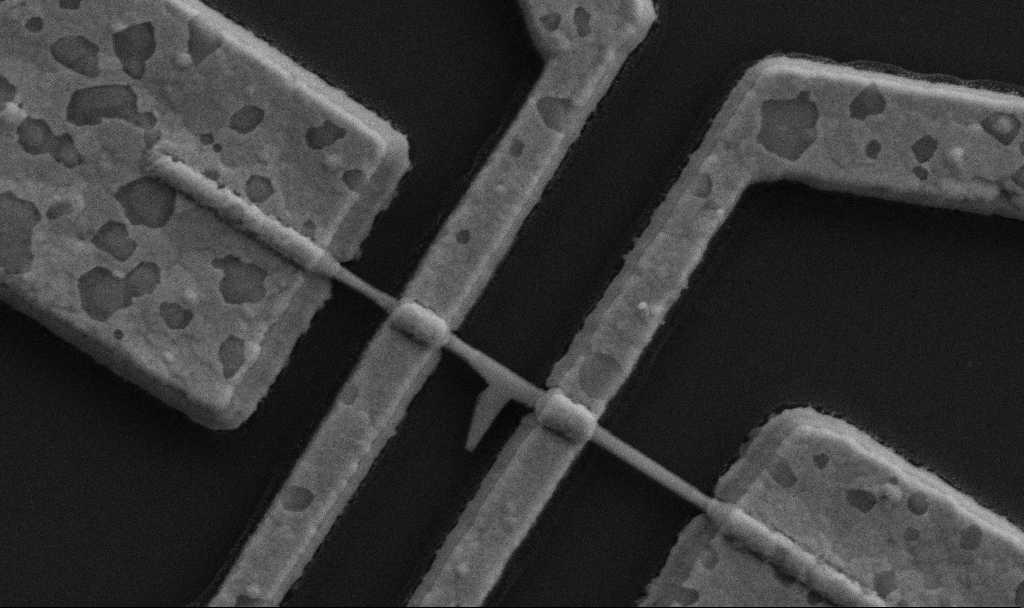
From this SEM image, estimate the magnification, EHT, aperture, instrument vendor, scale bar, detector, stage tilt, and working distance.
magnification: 60 K X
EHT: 5 kV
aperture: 30 µm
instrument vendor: Zeiss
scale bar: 1000 nm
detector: SE2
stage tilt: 0°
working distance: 9.7 mm